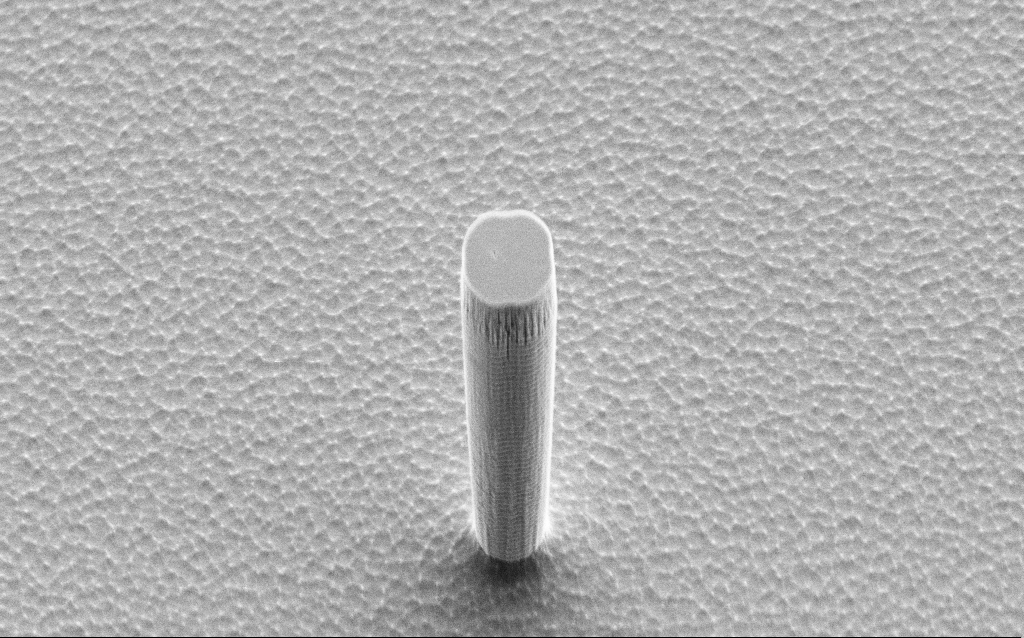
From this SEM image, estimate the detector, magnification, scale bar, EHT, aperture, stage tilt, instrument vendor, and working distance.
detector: SE2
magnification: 5.35 K X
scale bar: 10000 nm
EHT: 5 kV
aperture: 30 µm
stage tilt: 50°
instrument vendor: Zeiss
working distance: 10 mm